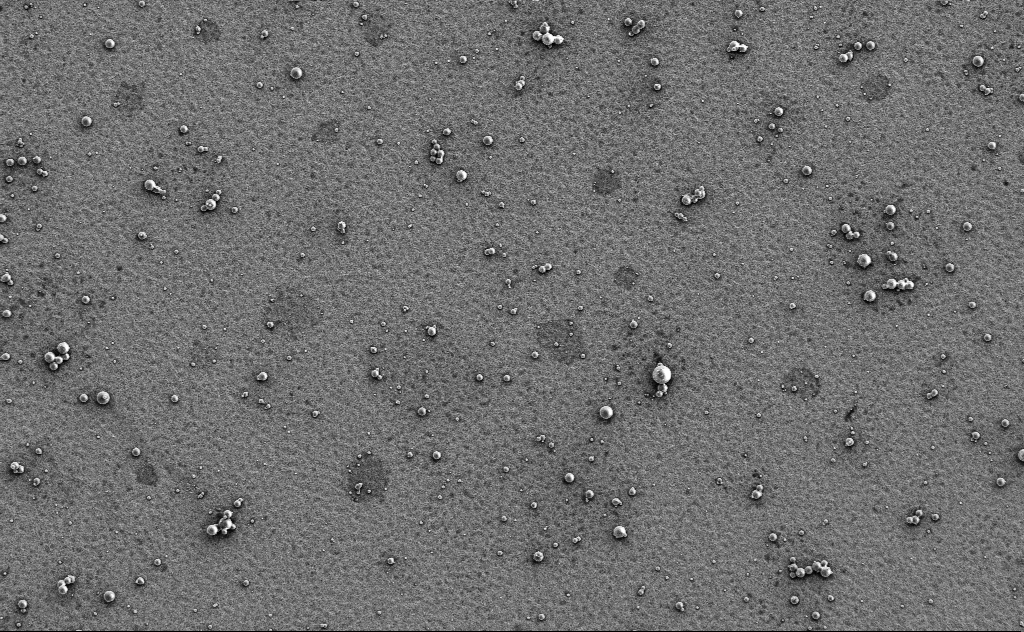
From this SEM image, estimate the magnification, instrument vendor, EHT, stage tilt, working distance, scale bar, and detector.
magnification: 2.44 K X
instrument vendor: Zeiss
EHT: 3 kV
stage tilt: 0°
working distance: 13 mm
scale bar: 10000 nm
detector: SE2